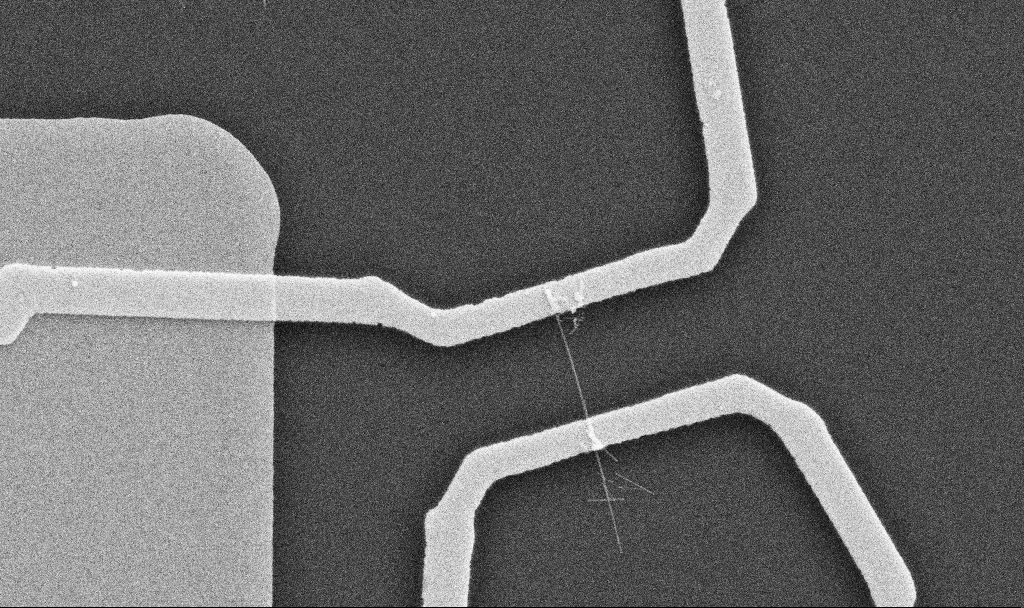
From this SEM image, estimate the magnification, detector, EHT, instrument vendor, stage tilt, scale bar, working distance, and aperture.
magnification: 20 K X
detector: SE2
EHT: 10 kV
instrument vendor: Zeiss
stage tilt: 0°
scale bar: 1000 nm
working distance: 10.7 mm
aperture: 30 µm